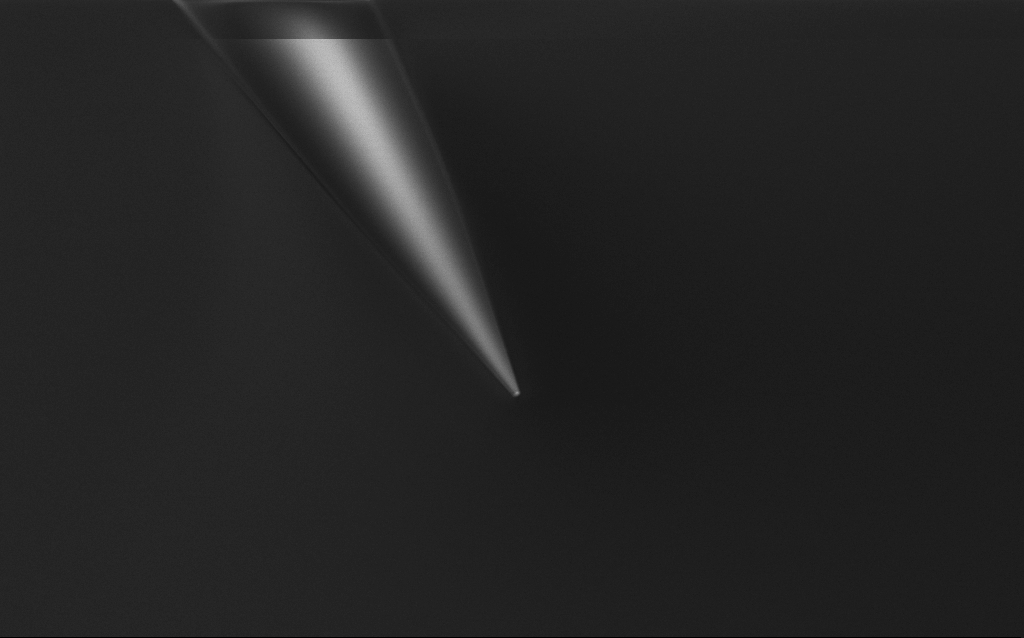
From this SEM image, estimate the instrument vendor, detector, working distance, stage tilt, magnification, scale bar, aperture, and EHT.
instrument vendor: Zeiss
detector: InLens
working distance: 7 mm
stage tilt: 45°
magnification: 1 K X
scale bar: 20000 nm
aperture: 30 µm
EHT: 1 kV